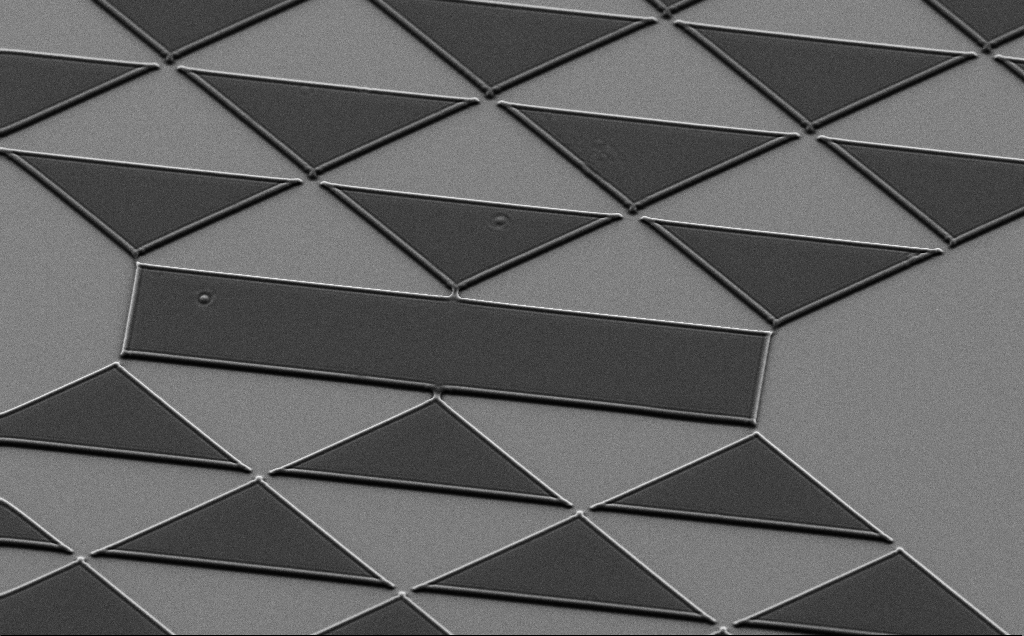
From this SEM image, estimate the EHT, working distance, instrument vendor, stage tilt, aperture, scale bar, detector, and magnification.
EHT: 7 kV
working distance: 5 mm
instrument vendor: Zeiss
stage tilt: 35°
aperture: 30 µm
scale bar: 10000 nm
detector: SE2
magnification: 1.28 K X